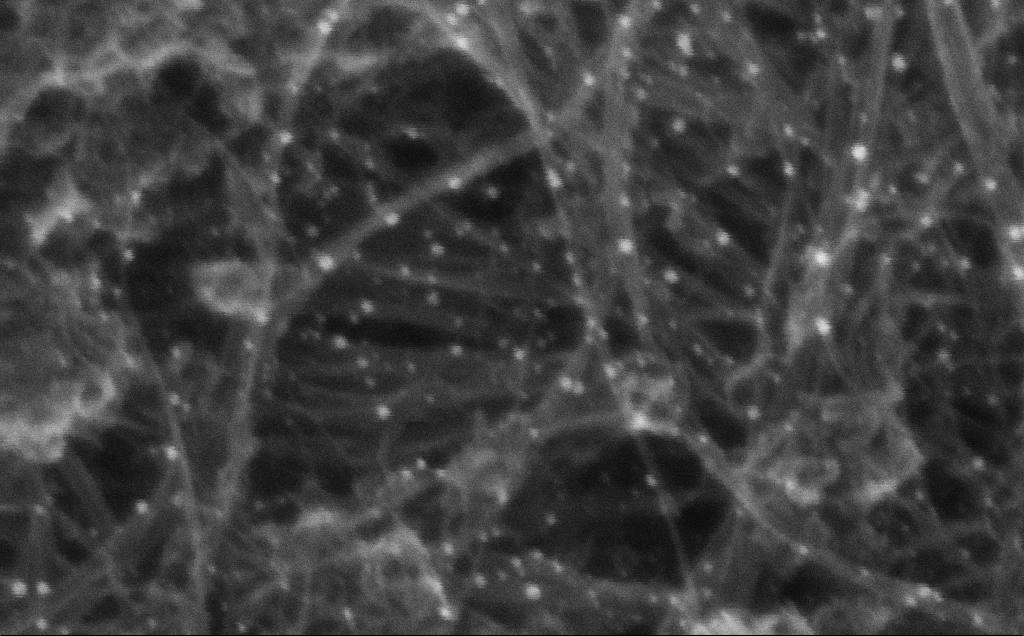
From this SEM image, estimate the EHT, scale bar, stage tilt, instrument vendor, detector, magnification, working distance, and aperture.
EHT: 10 kV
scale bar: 100 nm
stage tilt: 0°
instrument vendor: Zeiss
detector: InLens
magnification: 430.52 K X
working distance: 3 mm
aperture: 30 µm